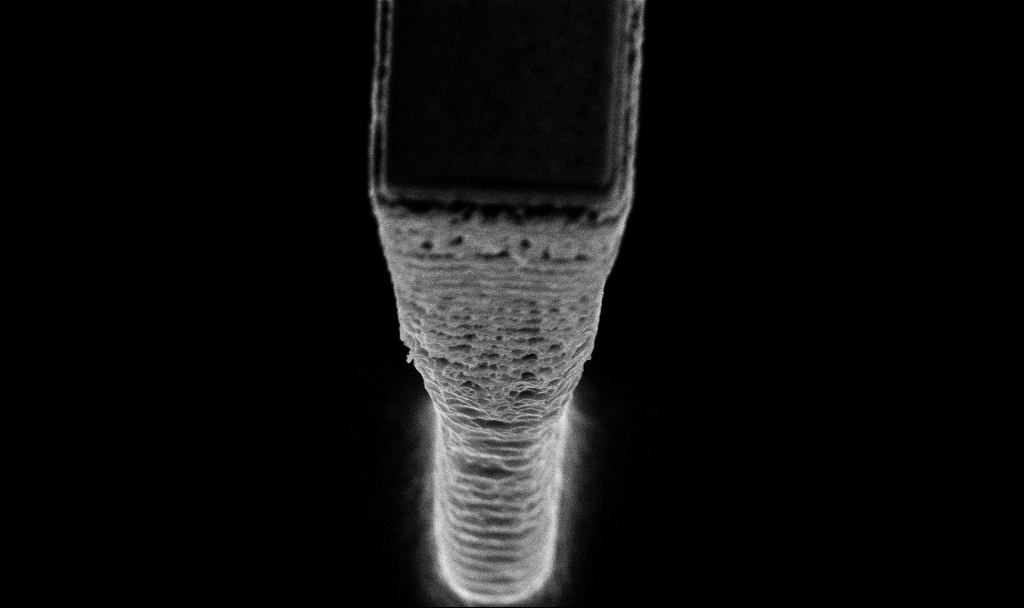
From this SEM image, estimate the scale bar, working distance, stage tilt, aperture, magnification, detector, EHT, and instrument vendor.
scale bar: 1000 nm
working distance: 4.1 mm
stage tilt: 20.2°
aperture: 30 µm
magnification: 29.16 K X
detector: InLens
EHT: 5 kV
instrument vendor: Zeiss